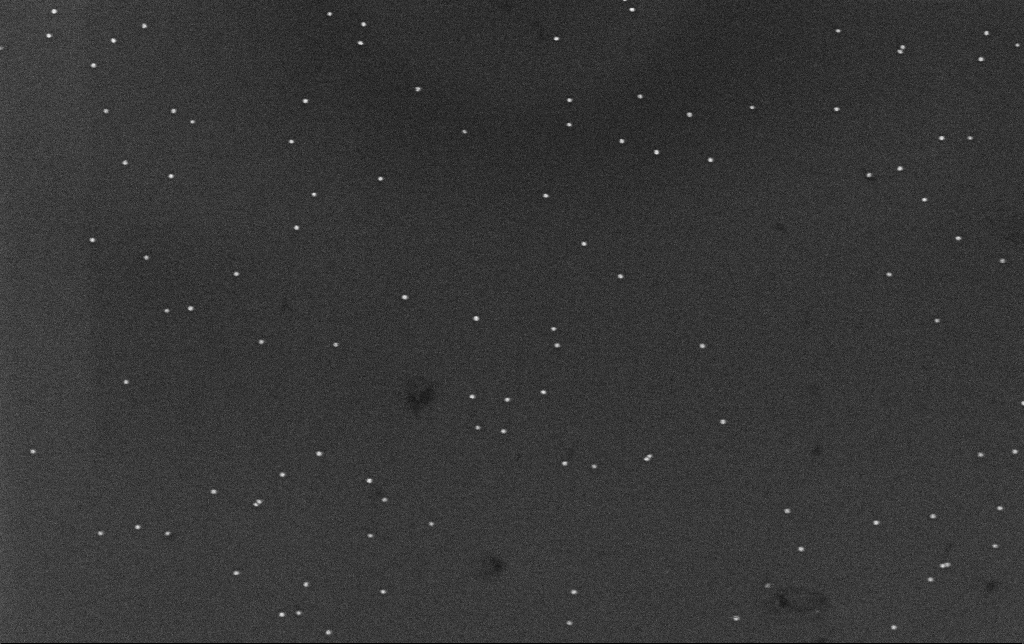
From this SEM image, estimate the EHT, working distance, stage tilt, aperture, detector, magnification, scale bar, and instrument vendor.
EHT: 10 kV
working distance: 3.1 mm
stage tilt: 0°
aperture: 30 µm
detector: InLens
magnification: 100 K X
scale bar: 200 nm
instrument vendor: Zeiss